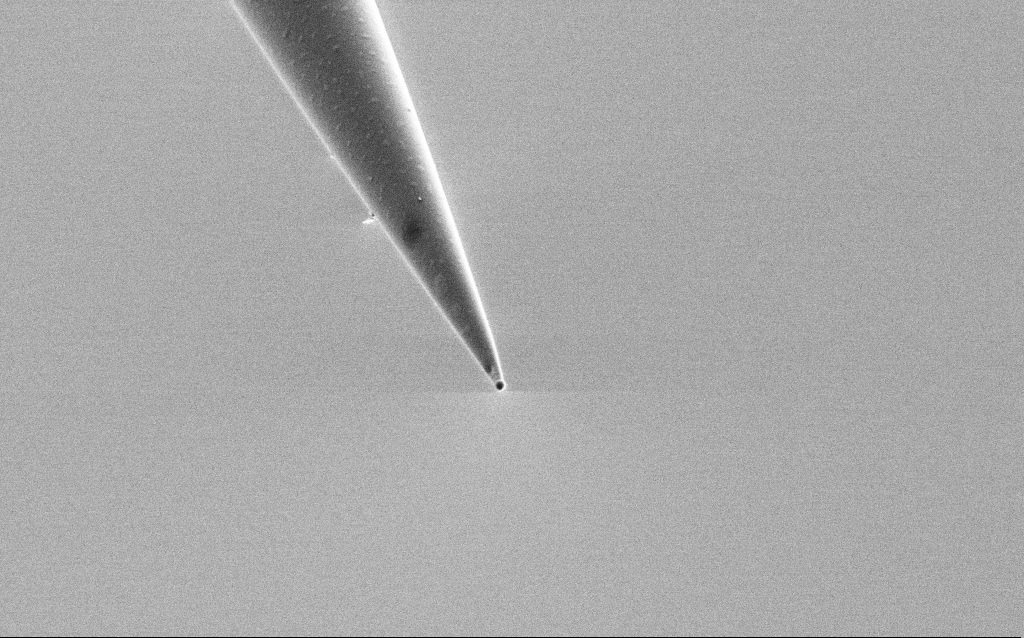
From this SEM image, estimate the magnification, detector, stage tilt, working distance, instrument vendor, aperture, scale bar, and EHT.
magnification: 25 K X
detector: SE2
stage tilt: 45°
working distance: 7.6 mm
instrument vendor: Zeiss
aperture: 30 µm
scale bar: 2000 nm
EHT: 1 kV